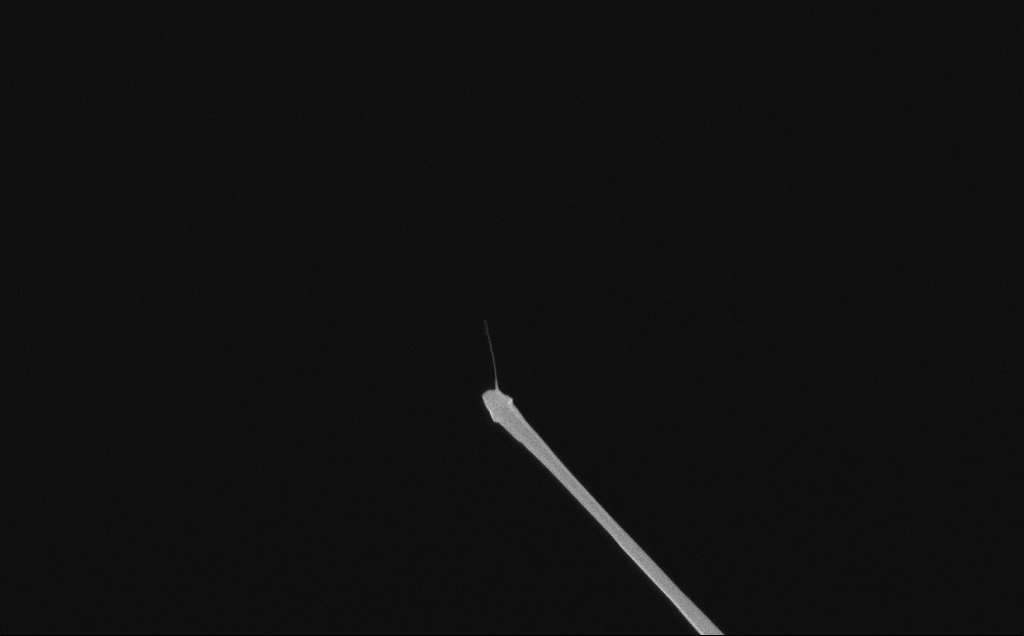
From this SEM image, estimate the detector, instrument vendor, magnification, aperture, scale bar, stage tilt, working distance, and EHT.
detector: InLens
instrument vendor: Zeiss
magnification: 45.95 K X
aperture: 30 µm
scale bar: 1000 nm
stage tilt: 0°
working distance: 6 mm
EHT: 10 kV